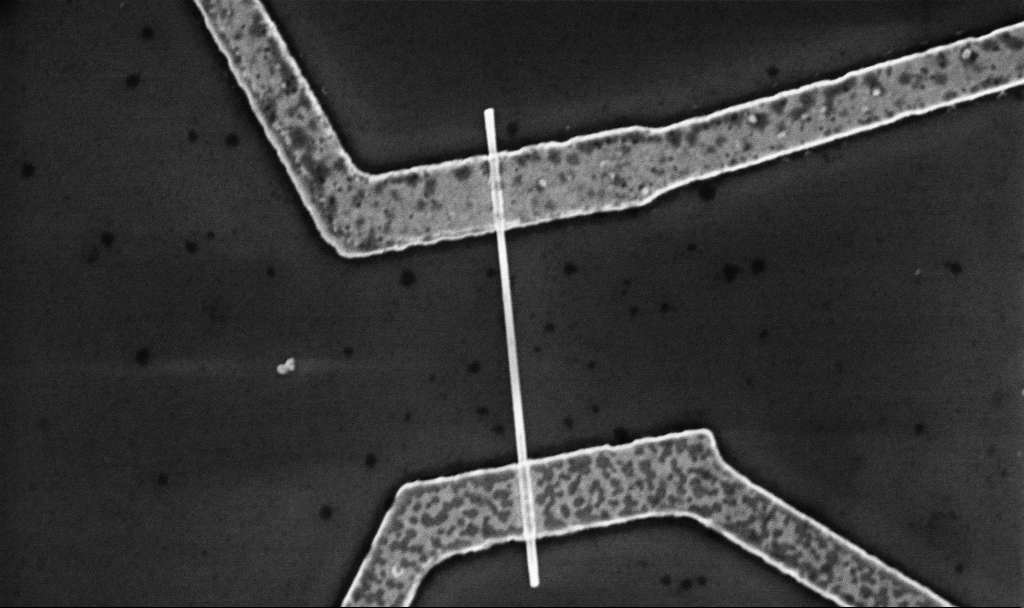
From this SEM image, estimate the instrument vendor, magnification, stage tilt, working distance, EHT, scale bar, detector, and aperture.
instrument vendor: Zeiss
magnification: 30 K X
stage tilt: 0°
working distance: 8.7 mm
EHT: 5 kV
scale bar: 1000 nm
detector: InLens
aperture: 30 µm